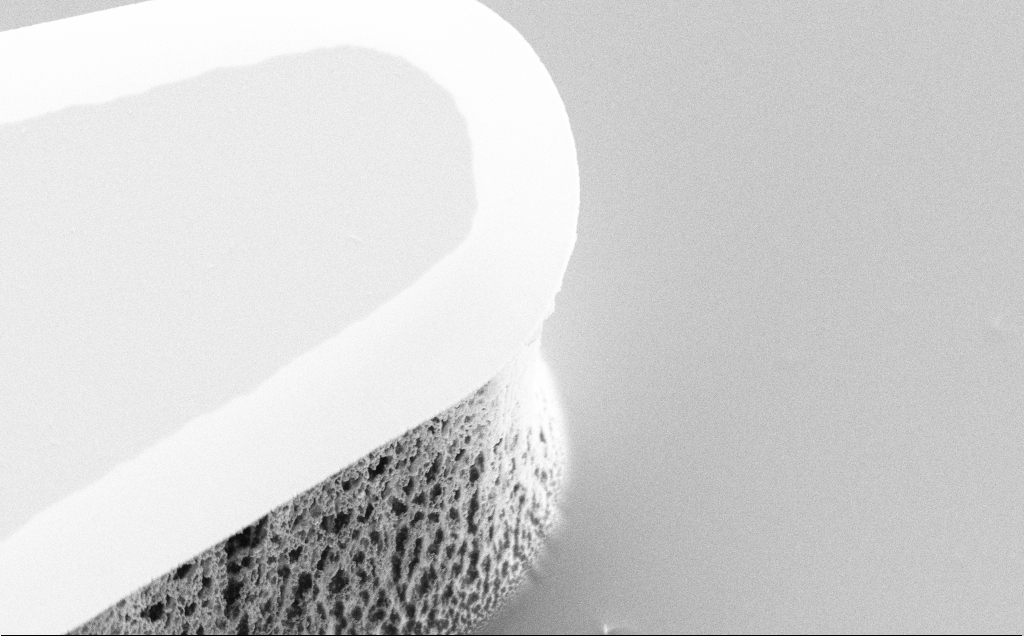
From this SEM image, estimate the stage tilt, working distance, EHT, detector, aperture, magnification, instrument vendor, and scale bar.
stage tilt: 45°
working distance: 8 mm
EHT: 5 kV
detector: SE2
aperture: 30 µm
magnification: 7.94 K X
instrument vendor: Zeiss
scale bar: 2000 nm